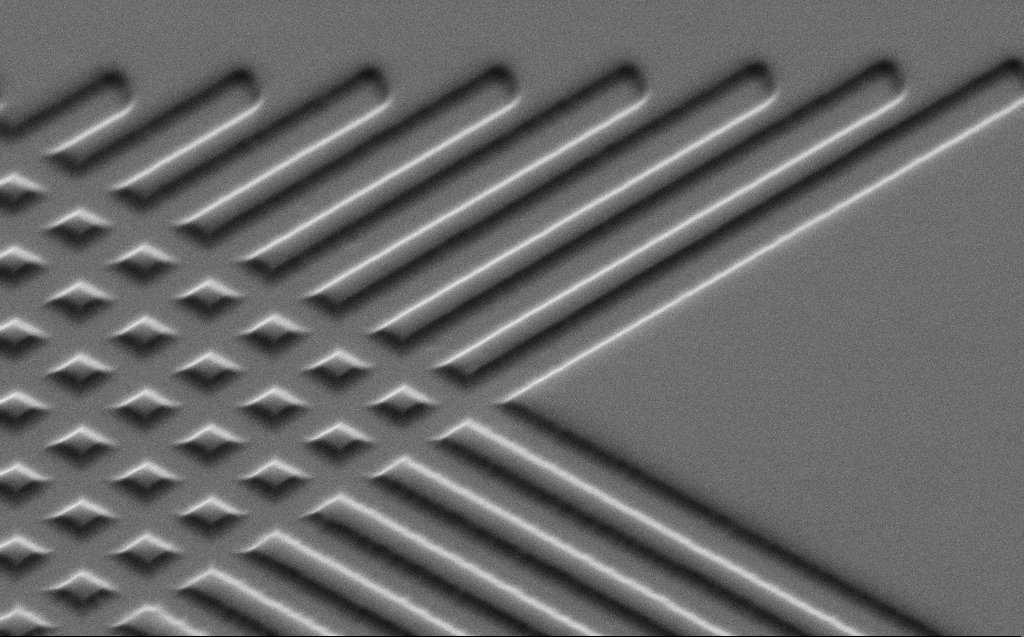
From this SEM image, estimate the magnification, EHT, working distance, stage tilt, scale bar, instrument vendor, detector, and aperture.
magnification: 9.47 K X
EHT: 3 kV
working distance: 6 mm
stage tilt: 45°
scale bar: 2000 nm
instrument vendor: Zeiss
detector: SE2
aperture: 30 µm